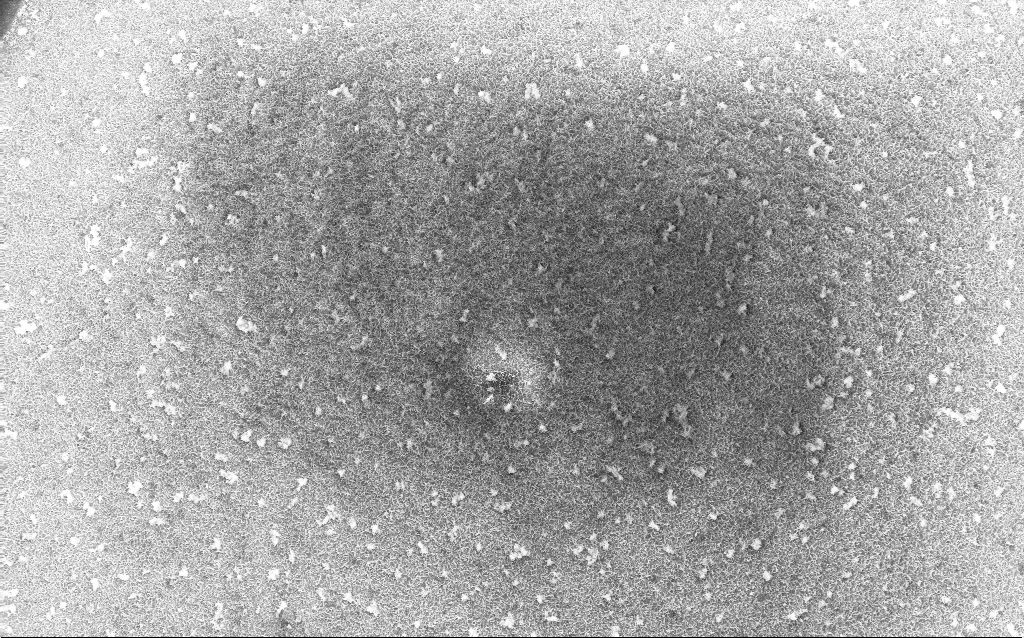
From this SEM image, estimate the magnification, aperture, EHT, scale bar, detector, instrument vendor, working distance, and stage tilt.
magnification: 1 K X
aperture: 30 µm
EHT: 10 kV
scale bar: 20000 nm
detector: InLens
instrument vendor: Zeiss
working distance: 2.8 mm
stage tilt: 0°